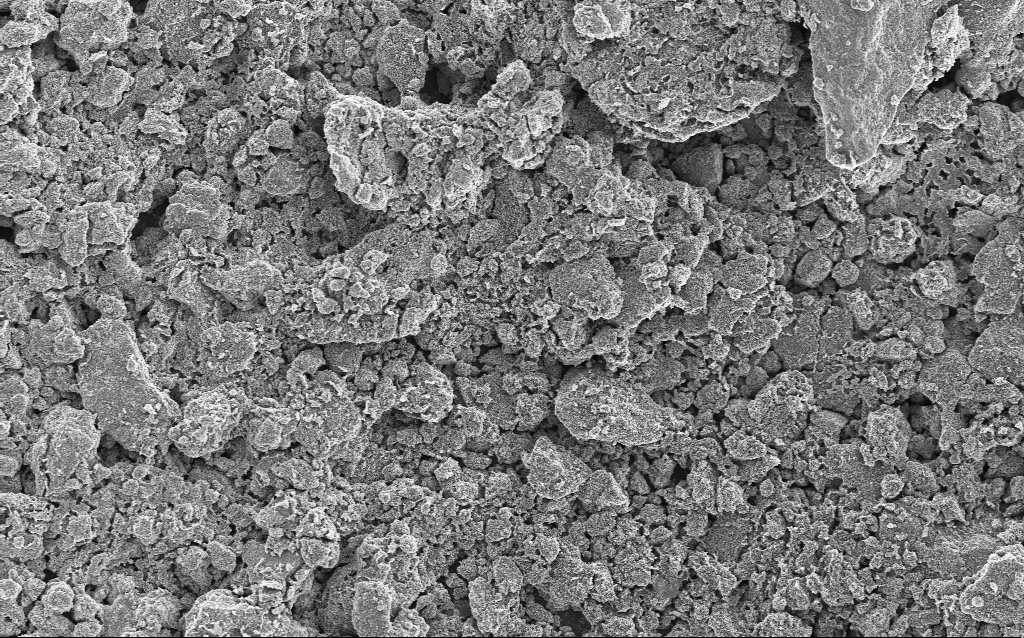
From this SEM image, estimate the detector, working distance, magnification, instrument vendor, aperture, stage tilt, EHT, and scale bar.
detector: InLens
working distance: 4.4 mm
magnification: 1.23 K X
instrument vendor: Zeiss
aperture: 30 µm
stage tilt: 0°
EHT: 5 kV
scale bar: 20000 nm